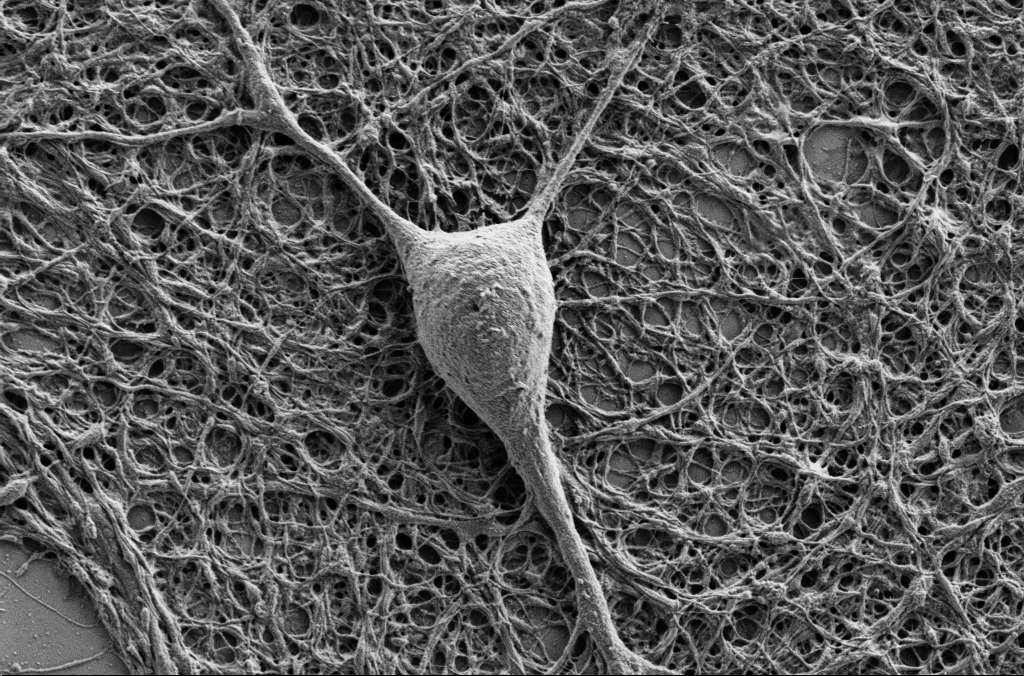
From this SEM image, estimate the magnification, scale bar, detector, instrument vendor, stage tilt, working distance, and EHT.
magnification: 10 K X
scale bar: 2000 nm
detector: SE2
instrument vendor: Zeiss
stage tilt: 0°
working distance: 4.1 mm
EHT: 1 kV